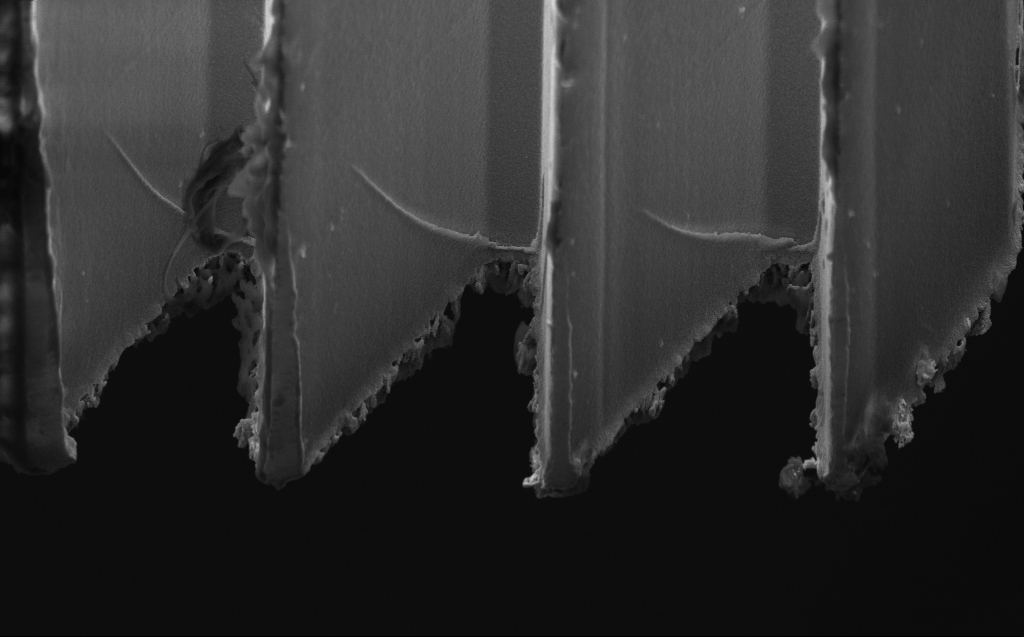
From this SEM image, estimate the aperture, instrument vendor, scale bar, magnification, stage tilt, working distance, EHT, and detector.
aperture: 30 µm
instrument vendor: Zeiss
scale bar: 2000 nm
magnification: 9.05 K X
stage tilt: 45°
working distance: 5 mm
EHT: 5 kV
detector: InLens